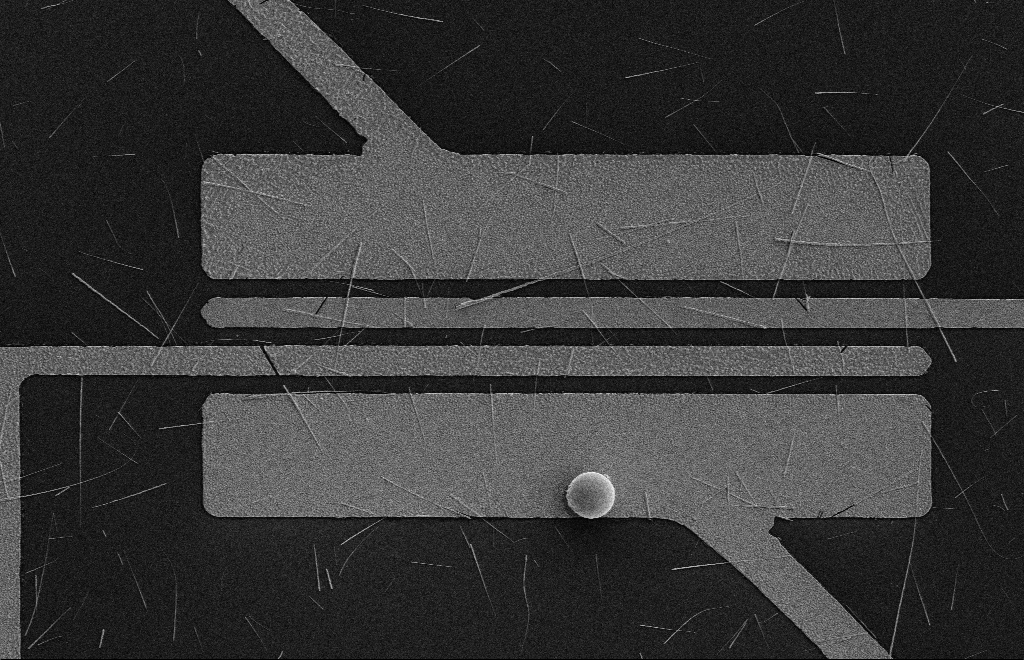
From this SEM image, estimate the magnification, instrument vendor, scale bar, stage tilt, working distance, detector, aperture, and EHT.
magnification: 4.44 K X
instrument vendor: Zeiss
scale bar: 10000 nm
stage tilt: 0°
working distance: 16 mm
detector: SE2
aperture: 10 µm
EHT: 5 kV